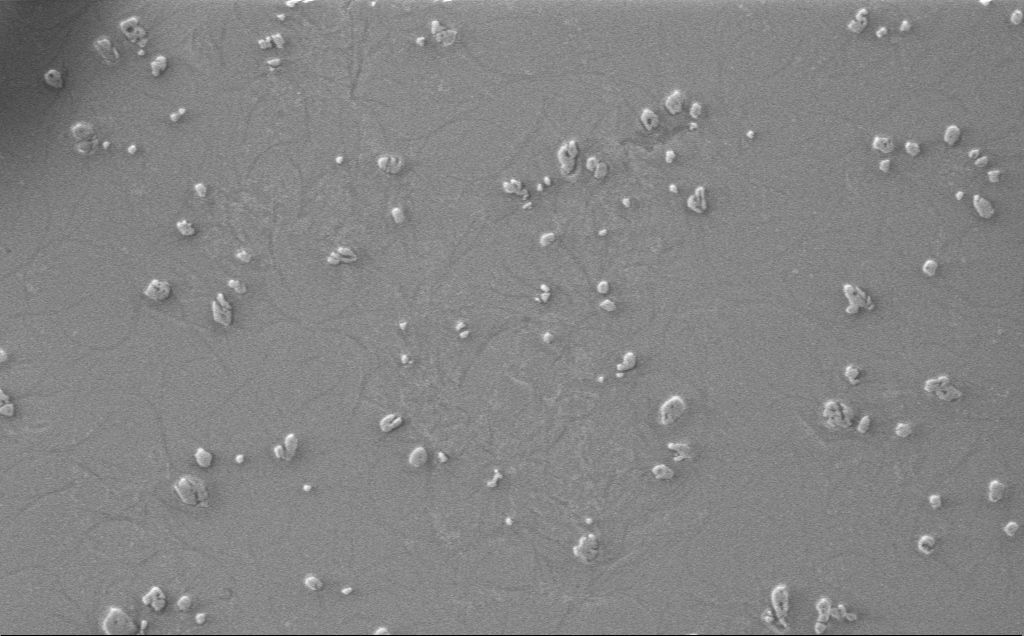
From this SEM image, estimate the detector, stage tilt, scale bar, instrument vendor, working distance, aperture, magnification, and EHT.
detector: InLens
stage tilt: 0°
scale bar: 10000 nm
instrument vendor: Zeiss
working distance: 4 mm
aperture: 30 µm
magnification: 5.98 K X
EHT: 1 kV